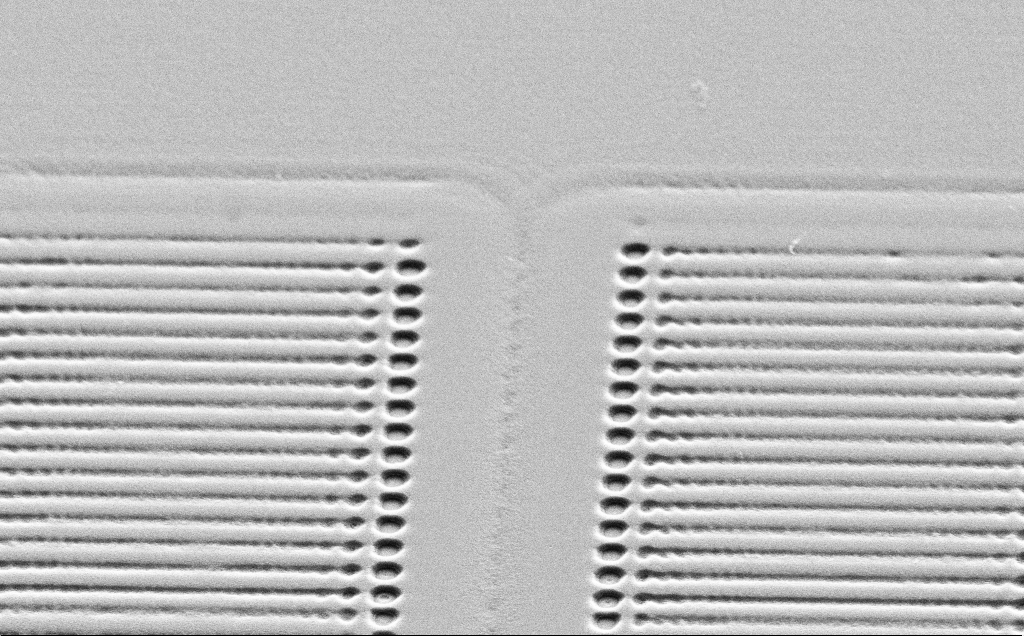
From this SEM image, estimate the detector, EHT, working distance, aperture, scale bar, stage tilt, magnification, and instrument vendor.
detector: SE2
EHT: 5 kV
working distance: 10 mm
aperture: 30 µm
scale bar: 10000 nm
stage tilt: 45°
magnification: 6.15 K X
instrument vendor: Zeiss